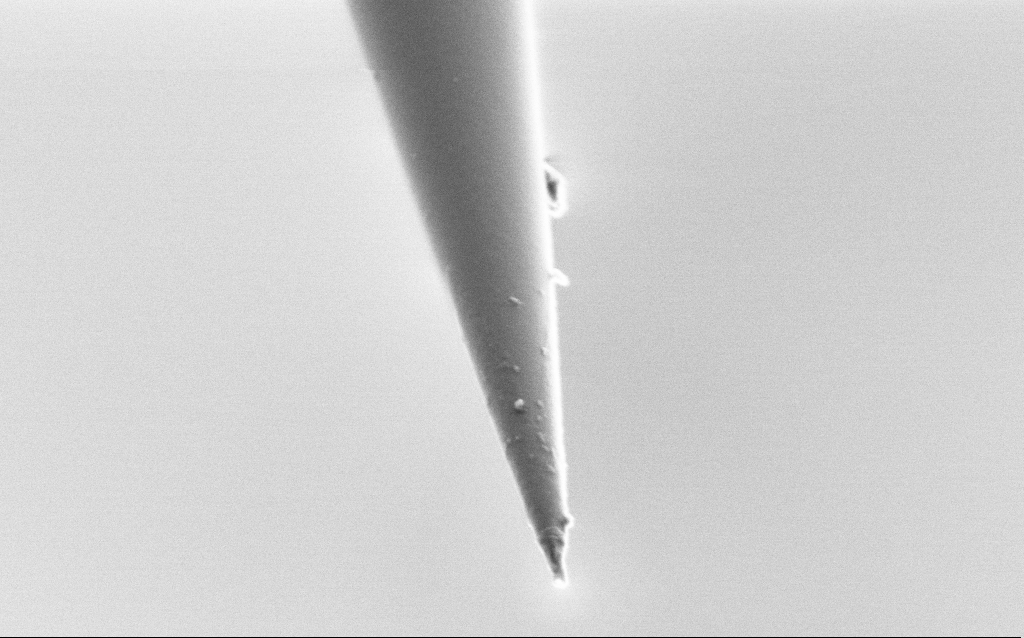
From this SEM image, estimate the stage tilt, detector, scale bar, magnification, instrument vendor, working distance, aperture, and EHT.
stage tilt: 45°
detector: SE2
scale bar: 1000 nm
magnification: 50 K X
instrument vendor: Zeiss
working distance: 6 mm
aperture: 30 µm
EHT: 1.5 kV